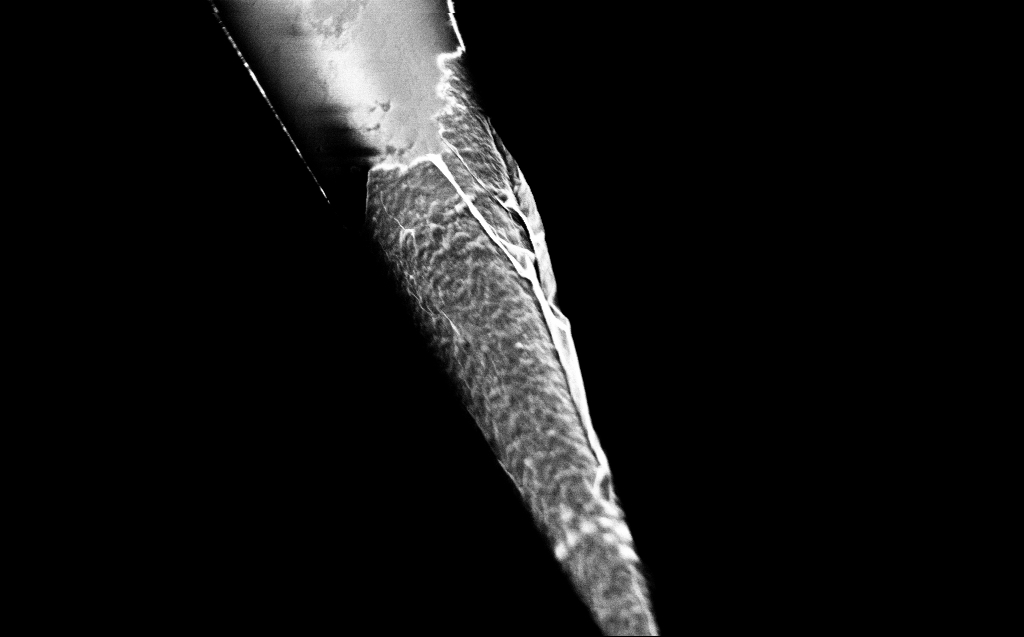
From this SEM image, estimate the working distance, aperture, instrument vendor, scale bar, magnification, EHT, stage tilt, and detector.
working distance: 3 mm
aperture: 30 µm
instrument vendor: Zeiss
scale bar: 2000 nm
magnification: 25 K X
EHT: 2 kV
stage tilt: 45°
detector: InLens